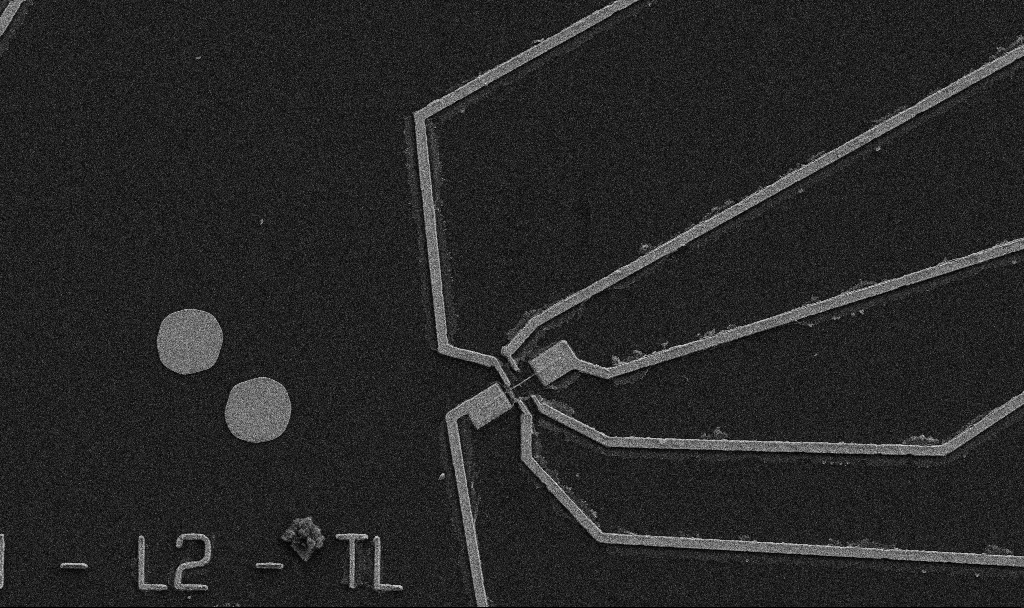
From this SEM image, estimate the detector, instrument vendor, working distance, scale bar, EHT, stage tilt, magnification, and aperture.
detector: SE2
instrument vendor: Zeiss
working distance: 10.7 mm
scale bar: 10000 nm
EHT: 5 kV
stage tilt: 0°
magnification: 5 K X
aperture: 30 µm